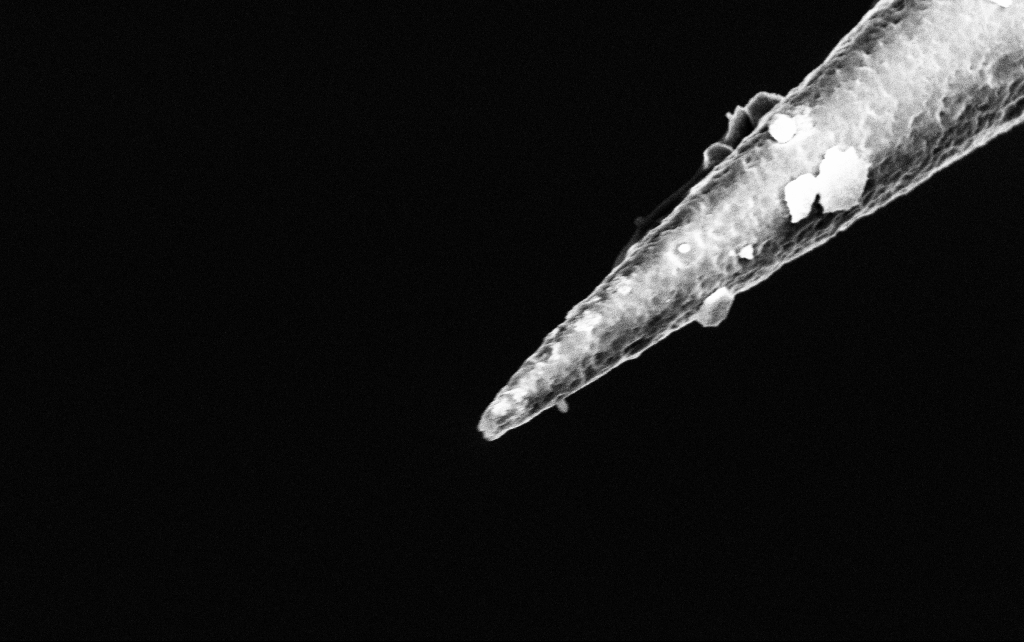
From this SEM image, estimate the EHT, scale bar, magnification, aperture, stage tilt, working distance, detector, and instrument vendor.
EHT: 3 kV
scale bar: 1000 nm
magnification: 50 K X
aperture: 30 µm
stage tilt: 45°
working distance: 7.6 mm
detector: InLens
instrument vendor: Zeiss